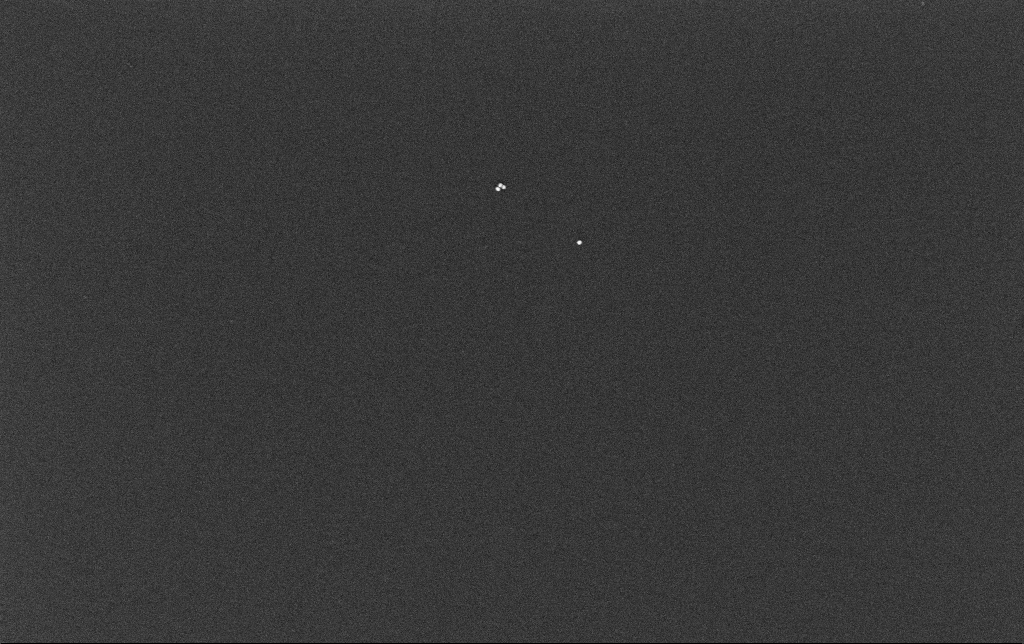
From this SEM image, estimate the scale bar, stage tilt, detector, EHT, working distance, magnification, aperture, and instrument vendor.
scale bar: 200 nm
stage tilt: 0°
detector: InLens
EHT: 10 kV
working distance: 3.4 mm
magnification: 100 K X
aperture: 30 µm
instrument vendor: Zeiss